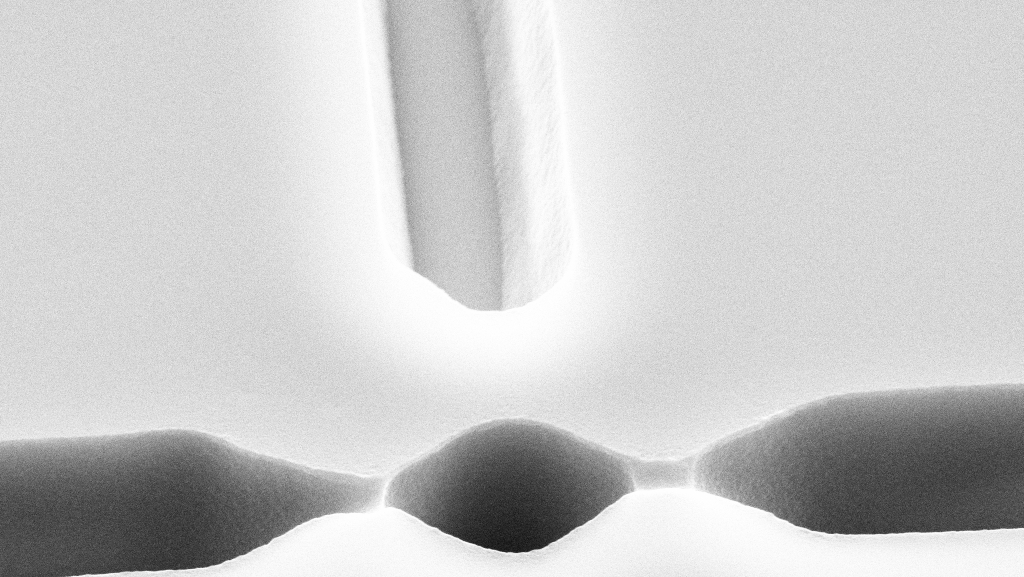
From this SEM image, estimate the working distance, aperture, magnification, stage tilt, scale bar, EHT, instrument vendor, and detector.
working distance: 13 mm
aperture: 30 µm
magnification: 29.14 K X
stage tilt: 45°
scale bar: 2000 nm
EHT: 10 kV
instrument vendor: Zeiss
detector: SE2